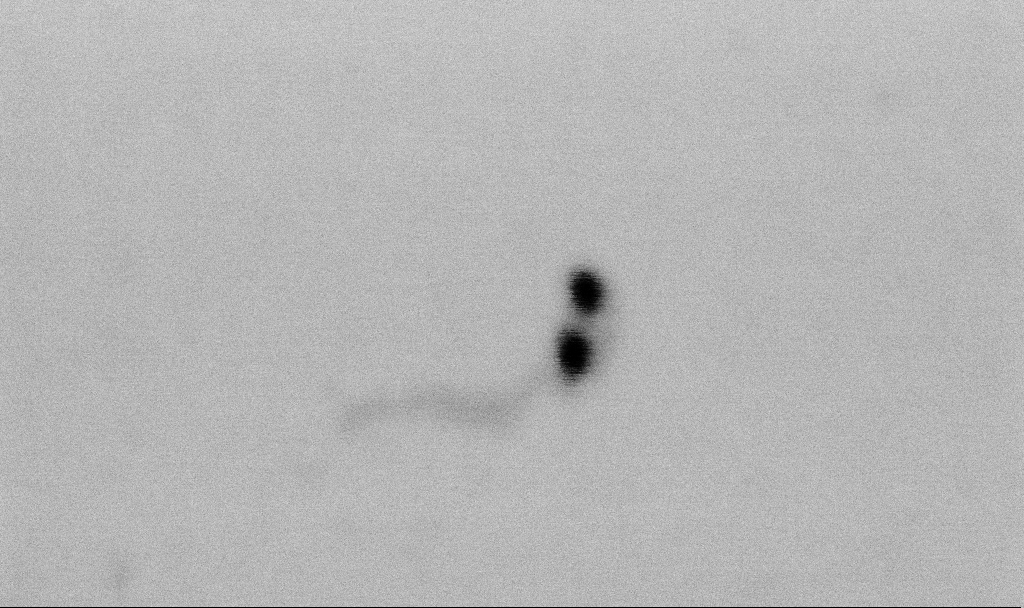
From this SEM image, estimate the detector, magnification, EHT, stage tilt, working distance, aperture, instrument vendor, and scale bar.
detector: SE2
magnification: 400 K X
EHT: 2 kV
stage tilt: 0°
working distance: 6.6 mm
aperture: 30 µm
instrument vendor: Zeiss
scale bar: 100 nm